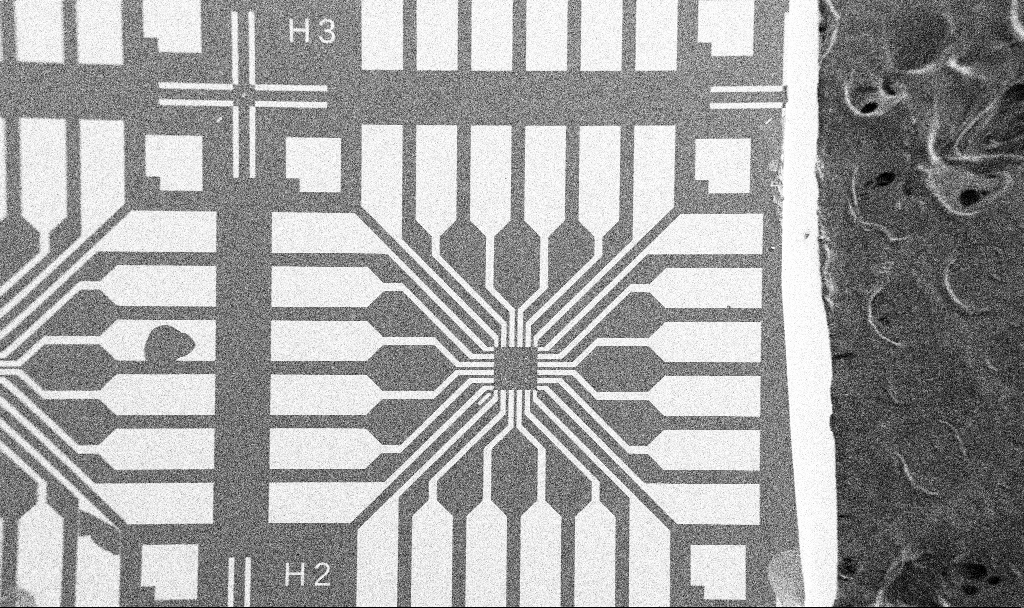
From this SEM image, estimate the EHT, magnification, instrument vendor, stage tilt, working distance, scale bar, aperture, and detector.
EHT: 5 kV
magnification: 0.1 K X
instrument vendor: Zeiss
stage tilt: -0°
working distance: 10.7 mm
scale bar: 200000 nm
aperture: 30 µm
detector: SE2